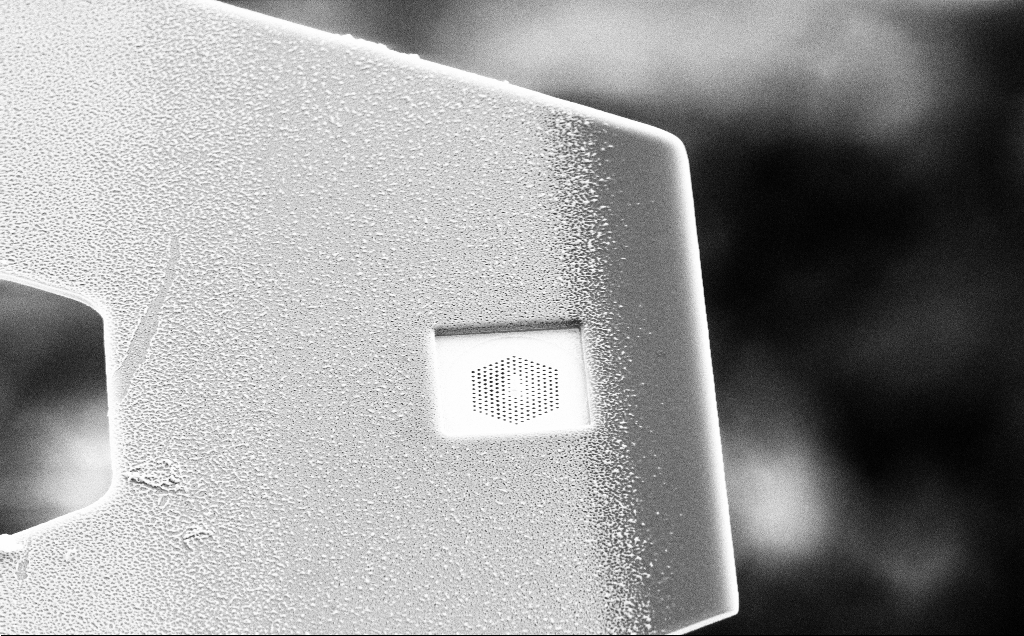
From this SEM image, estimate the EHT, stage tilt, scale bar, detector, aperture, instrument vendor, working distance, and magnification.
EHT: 10 kV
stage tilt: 45°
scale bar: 2000 nm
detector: SE2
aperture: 30 µm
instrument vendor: Zeiss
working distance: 11 mm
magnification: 8.75 K X